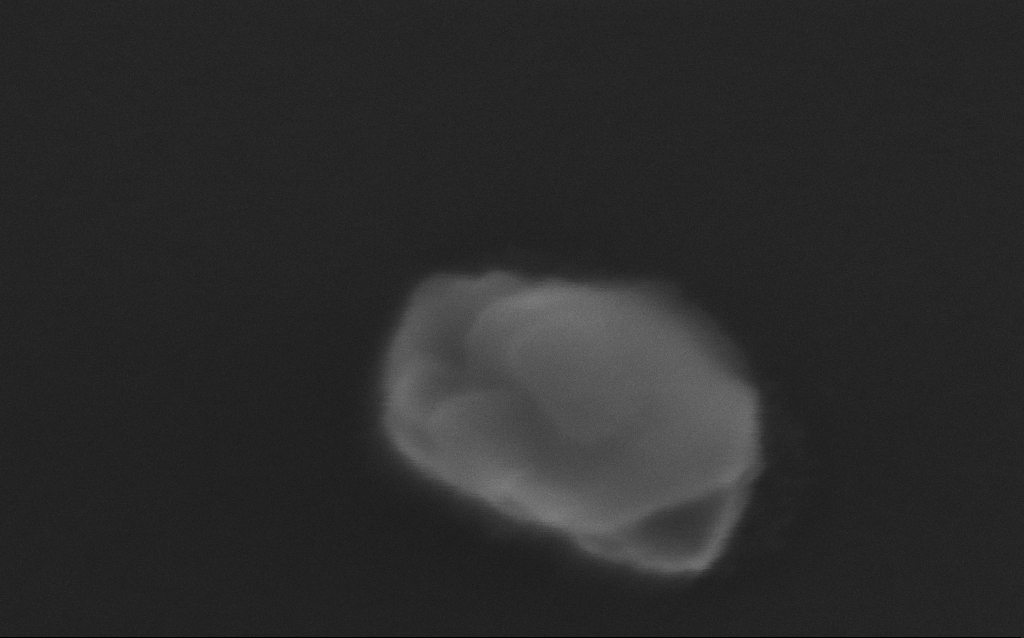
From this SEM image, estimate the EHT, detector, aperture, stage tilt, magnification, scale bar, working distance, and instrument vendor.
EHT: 10 kV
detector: InLens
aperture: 30 µm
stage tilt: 0°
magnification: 613.41 K X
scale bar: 100 nm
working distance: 5 mm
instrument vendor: Zeiss